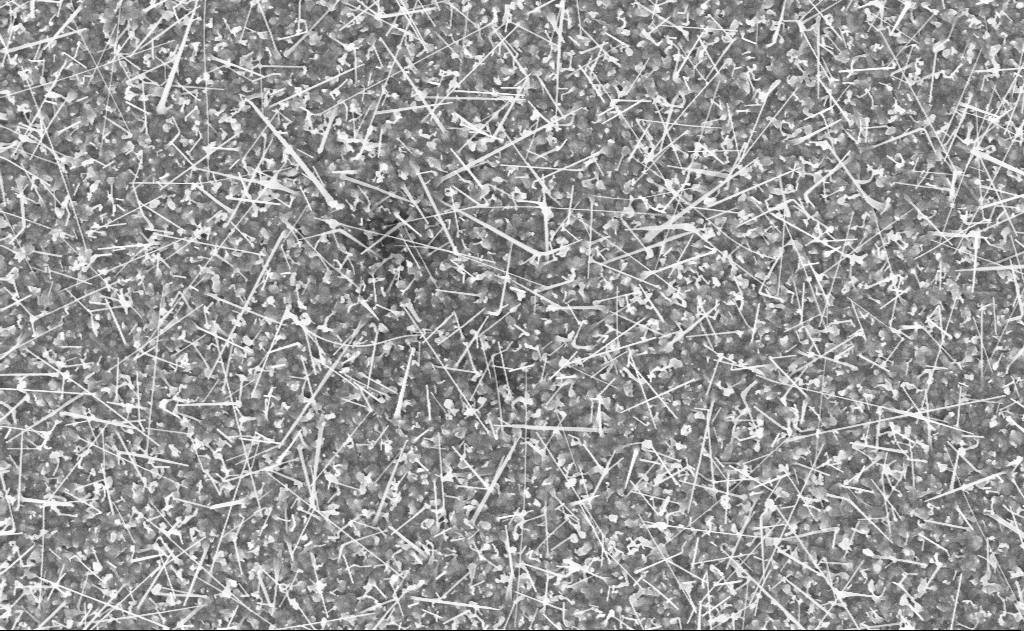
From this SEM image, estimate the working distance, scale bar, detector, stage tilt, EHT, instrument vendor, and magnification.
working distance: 20 mm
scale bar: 1000 nm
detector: InLens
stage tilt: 0°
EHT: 10 kV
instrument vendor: Zeiss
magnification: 20 K X